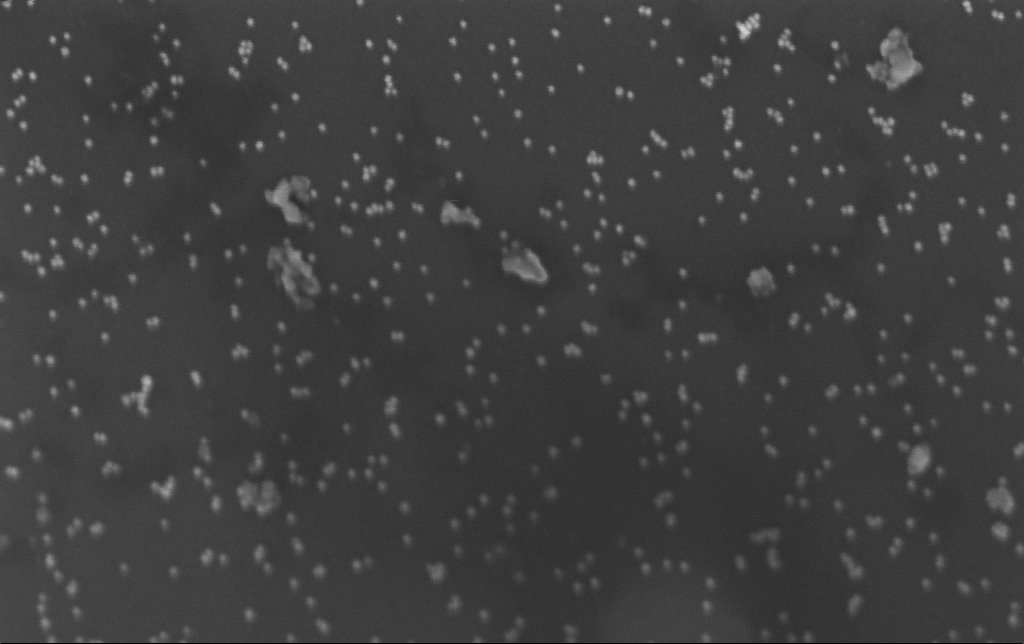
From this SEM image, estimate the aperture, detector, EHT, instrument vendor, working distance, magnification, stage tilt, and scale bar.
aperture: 30 µm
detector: InLens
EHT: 3 kV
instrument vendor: Zeiss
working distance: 3.2 mm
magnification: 173.4 K X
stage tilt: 0°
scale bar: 200 nm